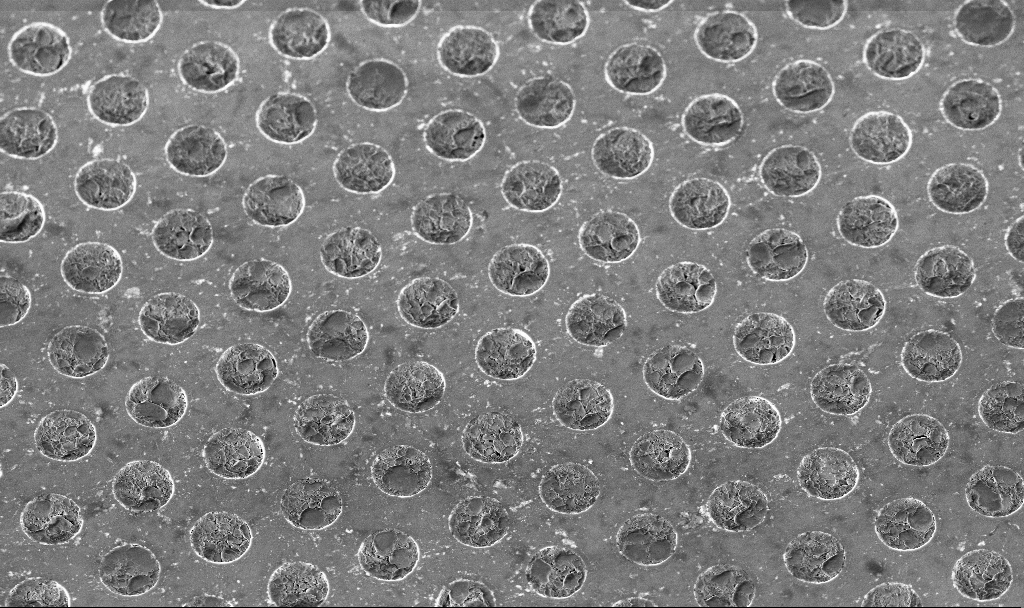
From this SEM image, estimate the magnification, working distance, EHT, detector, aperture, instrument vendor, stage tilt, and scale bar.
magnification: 6 K X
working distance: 4.5 mm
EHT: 3 kV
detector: InLens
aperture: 30 µm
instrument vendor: Zeiss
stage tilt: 30°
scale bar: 10000 nm